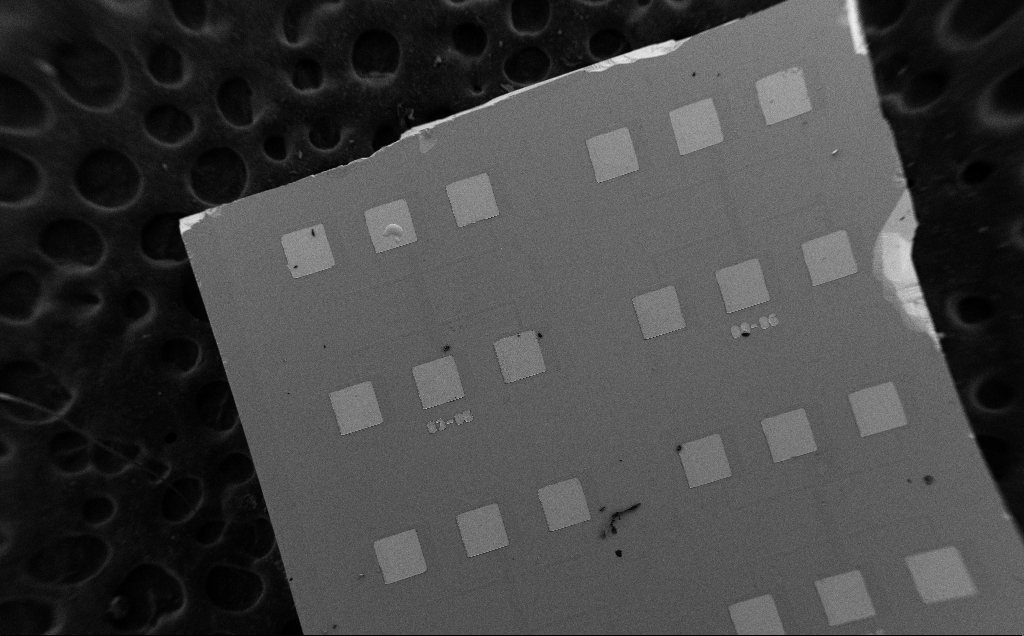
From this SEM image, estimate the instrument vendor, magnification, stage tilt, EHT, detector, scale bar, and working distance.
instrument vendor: Zeiss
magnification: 0.08 K X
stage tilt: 0°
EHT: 5 kV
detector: SE2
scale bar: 200000 nm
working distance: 6 mm